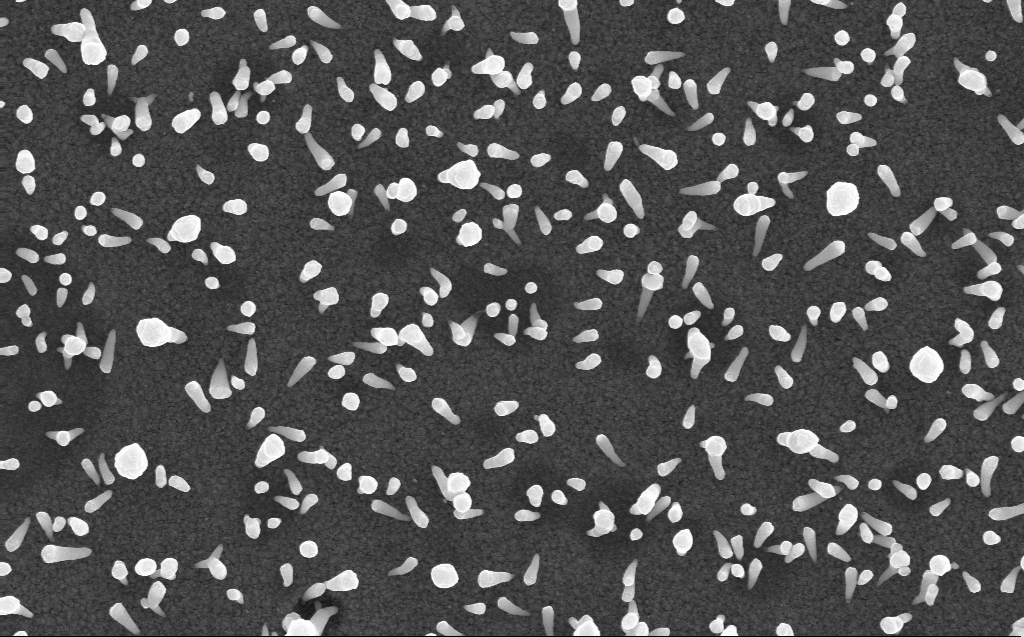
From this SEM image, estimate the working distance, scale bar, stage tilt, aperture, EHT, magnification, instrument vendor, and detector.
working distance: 3 mm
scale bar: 1000 nm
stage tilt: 0°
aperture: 30 µm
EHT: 10 kV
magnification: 42.08 K X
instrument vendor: Zeiss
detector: InLens